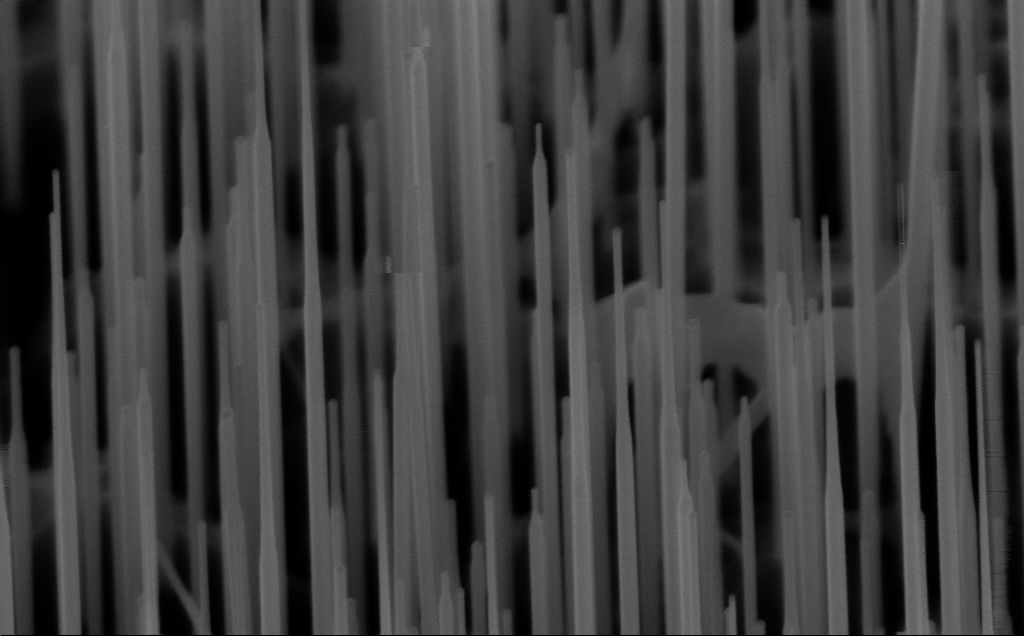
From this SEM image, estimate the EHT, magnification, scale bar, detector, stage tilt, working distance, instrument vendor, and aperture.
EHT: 10 kV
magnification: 80 K X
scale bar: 200 nm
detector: InLens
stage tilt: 45°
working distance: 6 mm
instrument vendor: Zeiss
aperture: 30 µm